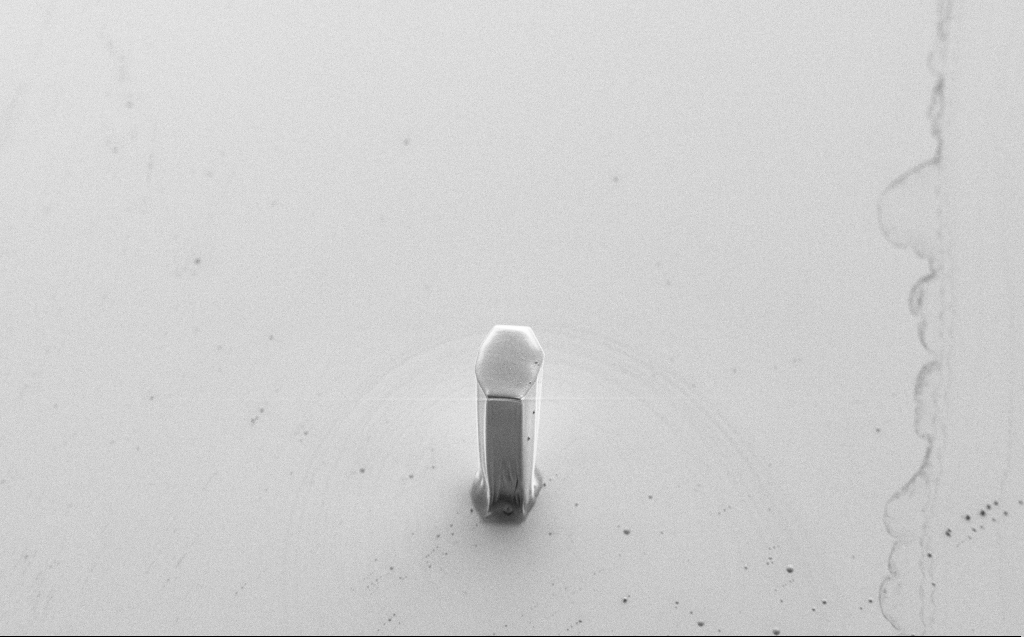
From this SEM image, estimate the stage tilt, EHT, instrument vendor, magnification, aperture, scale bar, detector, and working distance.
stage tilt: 45°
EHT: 2 kV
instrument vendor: Zeiss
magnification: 0.583 K X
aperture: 30 µm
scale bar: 100000 nm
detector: SE2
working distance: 8 mm